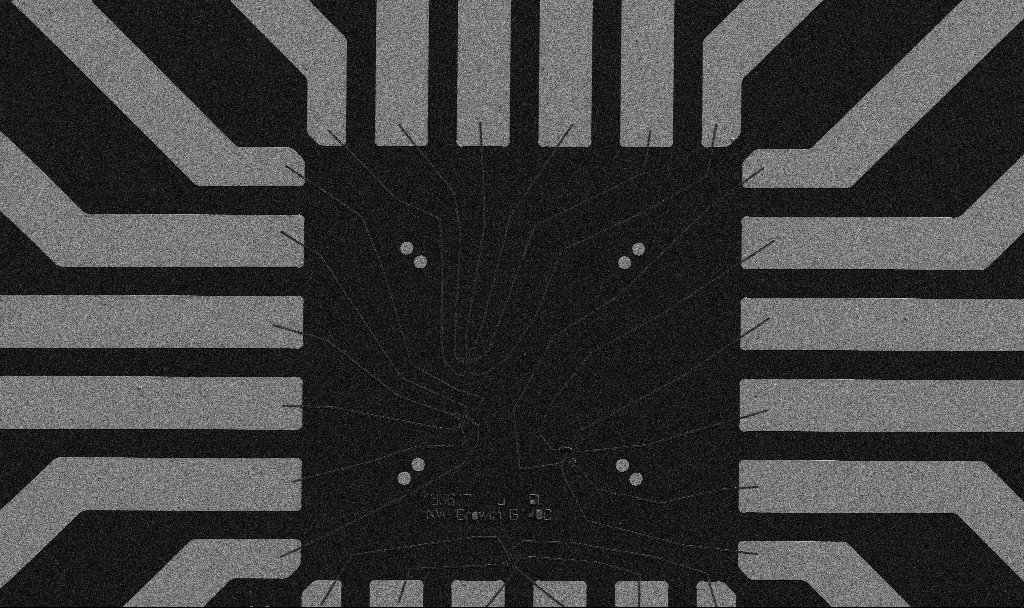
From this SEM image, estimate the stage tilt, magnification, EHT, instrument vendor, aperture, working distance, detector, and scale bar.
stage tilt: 0°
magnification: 1 K X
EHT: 5 kV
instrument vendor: Zeiss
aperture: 30 µm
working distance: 10.7 mm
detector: SE2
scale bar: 20000 nm